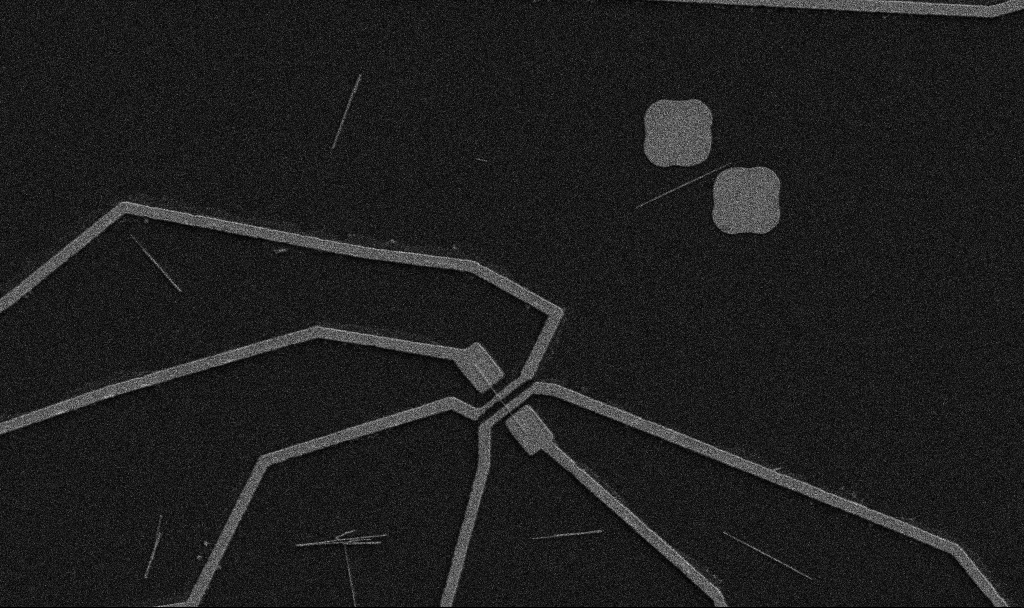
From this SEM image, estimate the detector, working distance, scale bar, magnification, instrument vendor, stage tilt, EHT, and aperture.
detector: SE2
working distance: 10.7 mm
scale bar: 10000 nm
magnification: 5 K X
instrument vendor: Zeiss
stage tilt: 0°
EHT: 5 kV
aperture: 30 µm